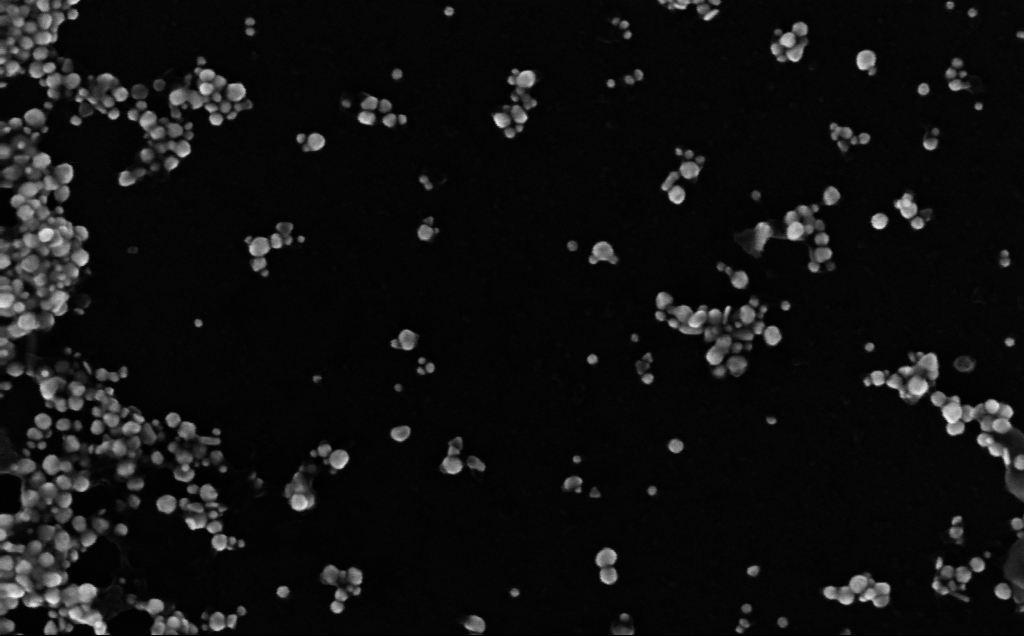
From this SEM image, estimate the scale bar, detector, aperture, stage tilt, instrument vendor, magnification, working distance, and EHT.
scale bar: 100 nm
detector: InLens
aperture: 30 µm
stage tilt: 0°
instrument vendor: Zeiss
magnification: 261.33 K X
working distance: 3 mm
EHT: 10 kV